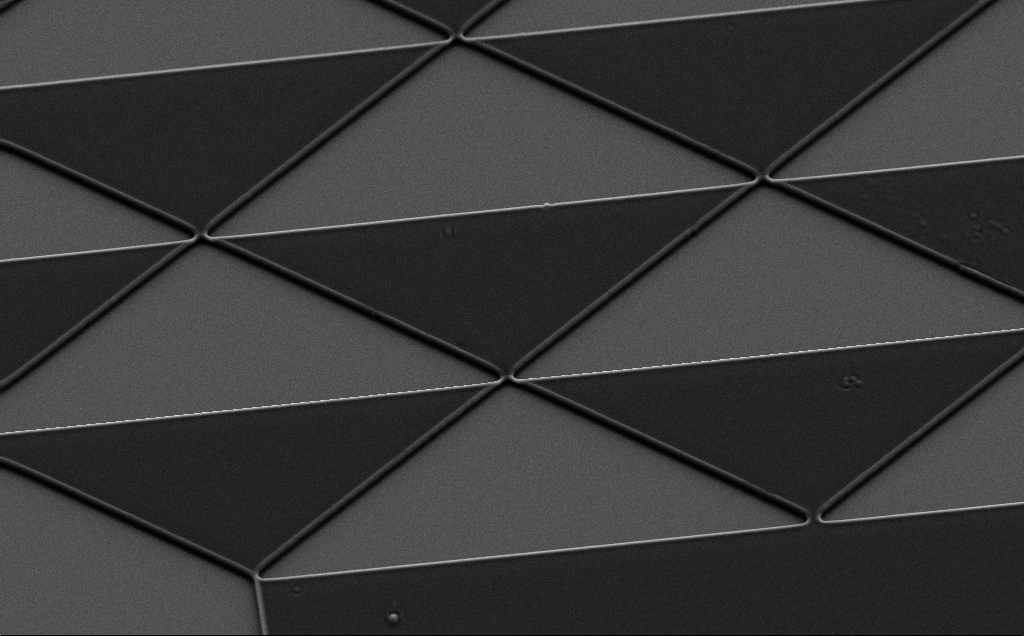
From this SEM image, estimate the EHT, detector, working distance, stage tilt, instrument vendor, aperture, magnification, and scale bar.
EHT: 7 kV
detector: SE2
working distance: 6 mm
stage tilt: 35°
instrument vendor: Zeiss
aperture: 30 µm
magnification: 2.23 K X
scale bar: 10000 nm